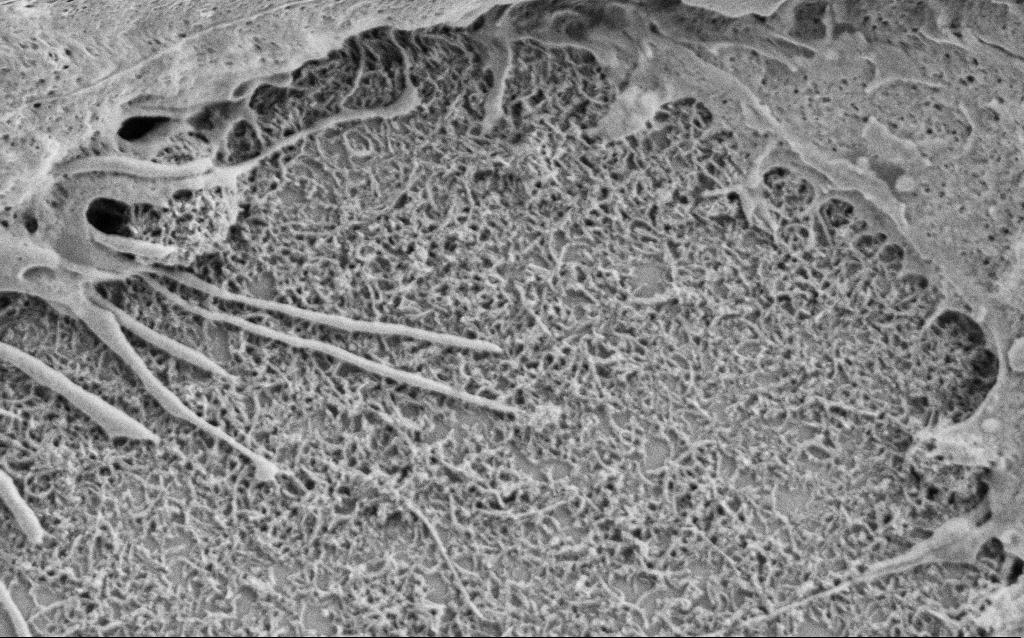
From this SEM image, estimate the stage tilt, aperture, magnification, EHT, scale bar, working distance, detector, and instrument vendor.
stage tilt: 0°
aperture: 30 µm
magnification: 25 K X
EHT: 1.5 kV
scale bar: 1000 nm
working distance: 6.8 mm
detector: SE2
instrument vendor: Zeiss